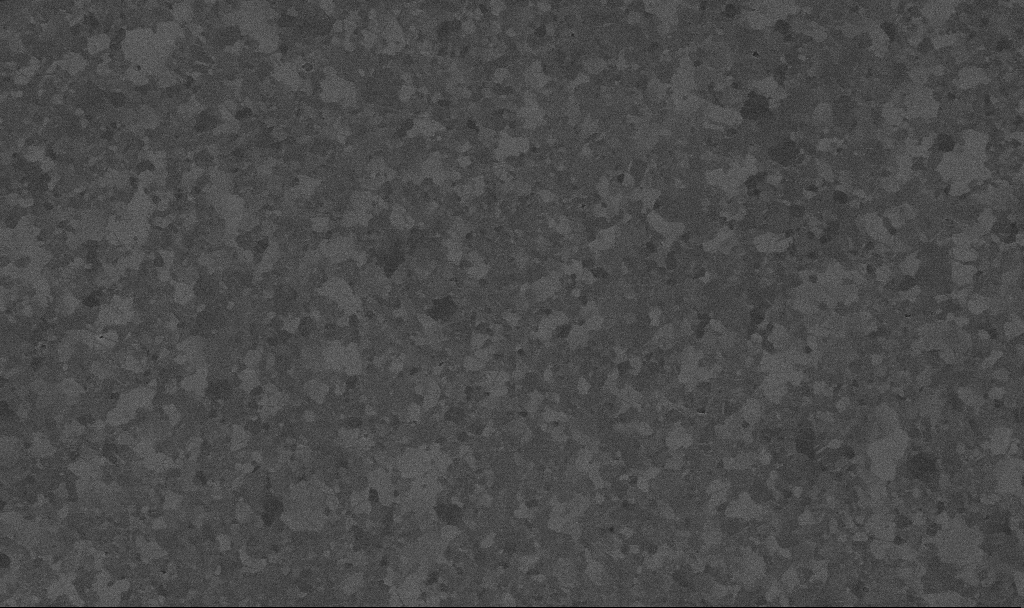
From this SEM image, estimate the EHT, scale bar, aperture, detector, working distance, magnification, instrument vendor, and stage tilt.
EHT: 10 kV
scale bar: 1000 nm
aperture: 30 µm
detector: InLens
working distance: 3.4 mm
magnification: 30 K X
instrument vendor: Zeiss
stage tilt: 0°